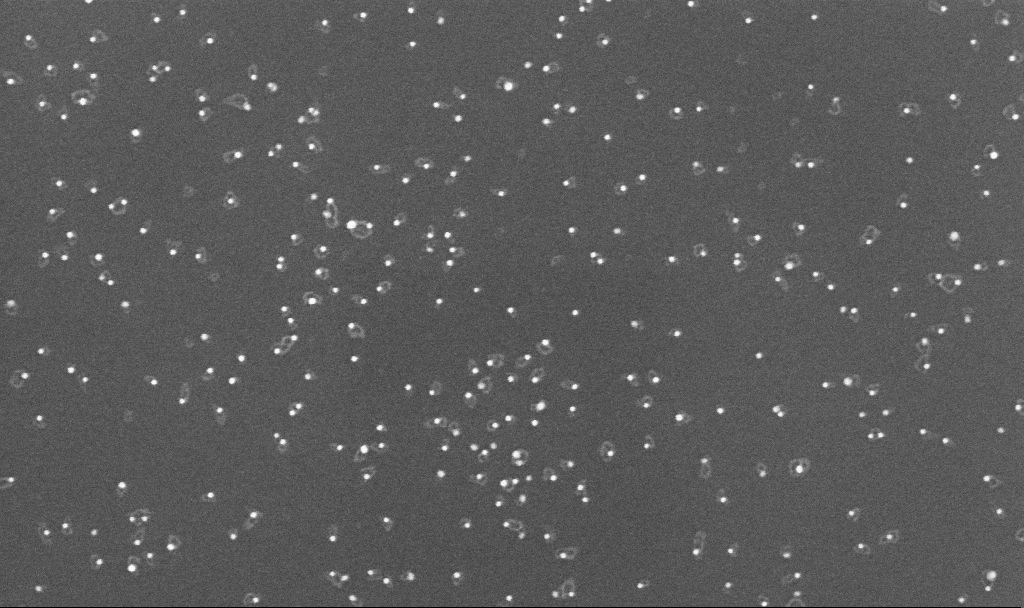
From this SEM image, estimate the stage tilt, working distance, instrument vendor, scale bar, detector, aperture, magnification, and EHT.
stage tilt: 0°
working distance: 3.4 mm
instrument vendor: Zeiss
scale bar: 100 nm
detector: InLens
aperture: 30 µm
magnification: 200 K X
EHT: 10 kV